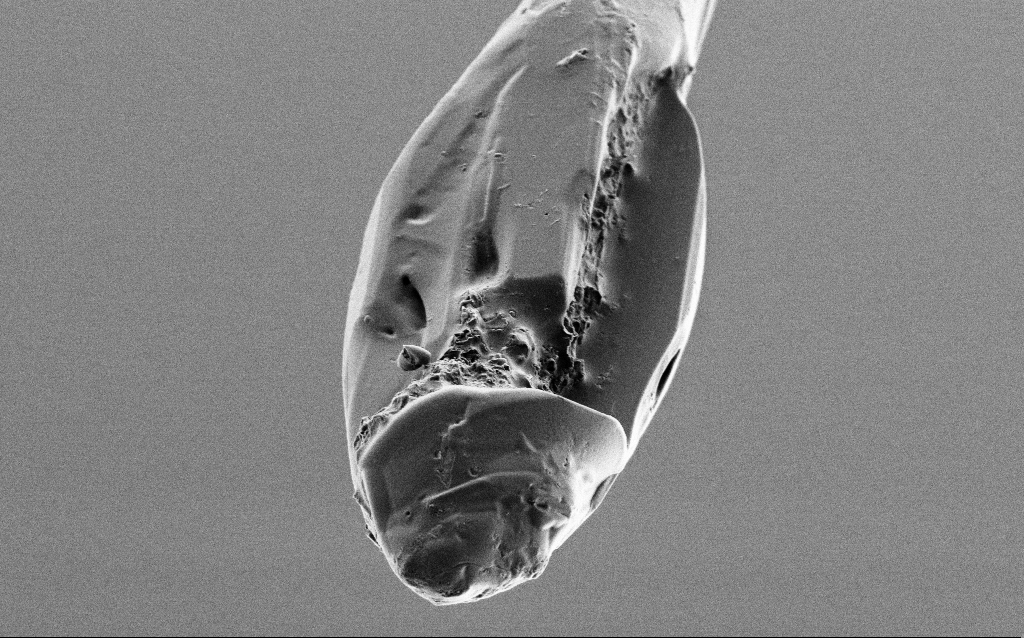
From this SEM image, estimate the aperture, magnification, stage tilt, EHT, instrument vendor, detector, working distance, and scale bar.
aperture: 30 µm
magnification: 10 K X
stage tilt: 45°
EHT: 1 kV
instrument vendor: Zeiss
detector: SE2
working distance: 6.5 mm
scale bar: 2000 nm